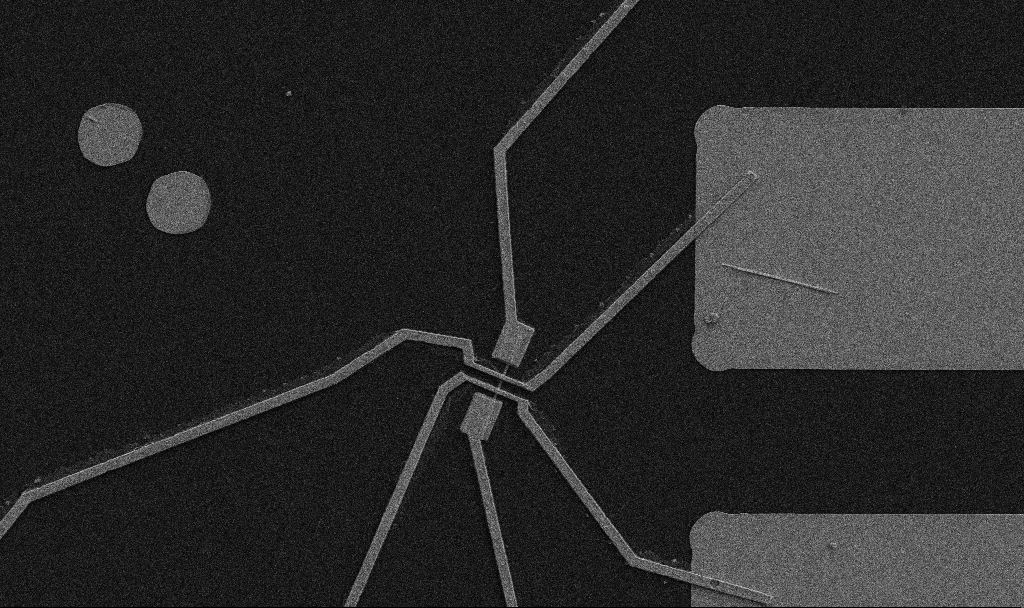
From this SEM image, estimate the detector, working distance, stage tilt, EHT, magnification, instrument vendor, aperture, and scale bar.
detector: SE2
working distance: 10.7 mm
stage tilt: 0°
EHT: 5 kV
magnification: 5 K X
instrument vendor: Zeiss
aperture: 30 µm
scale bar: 10000 nm